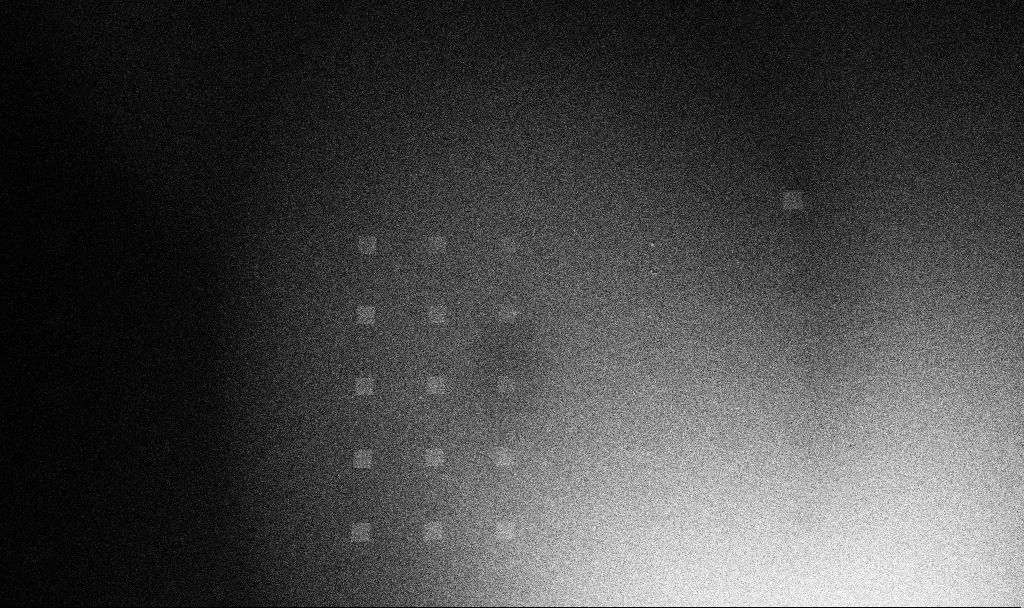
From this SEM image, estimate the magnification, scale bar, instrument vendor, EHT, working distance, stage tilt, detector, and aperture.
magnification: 0.067 K X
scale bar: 1e+06 nm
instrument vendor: Zeiss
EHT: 5 kV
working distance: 8.7 mm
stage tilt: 45°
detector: InLens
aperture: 30 µm